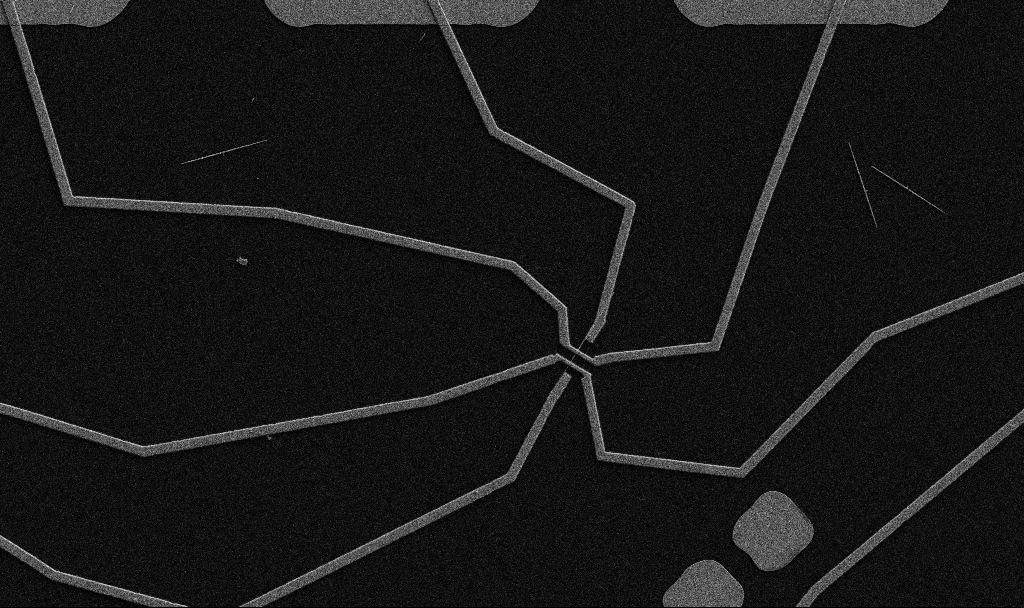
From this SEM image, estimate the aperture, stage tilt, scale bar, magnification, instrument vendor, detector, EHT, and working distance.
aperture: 30 µm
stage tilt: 0°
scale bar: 10000 nm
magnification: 5 K X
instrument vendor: Zeiss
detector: SE2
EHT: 5 kV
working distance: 10.7 mm